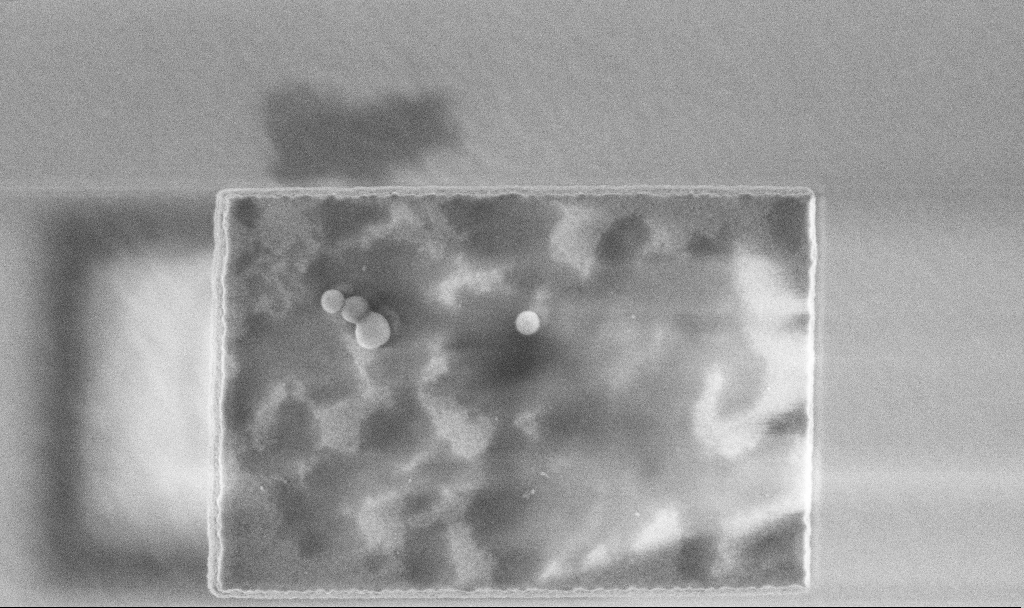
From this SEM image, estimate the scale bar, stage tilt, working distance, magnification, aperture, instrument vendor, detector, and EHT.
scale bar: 1000 nm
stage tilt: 0°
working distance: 3.3 mm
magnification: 49.45 K X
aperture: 30 µm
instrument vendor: Zeiss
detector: InLens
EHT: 3 kV